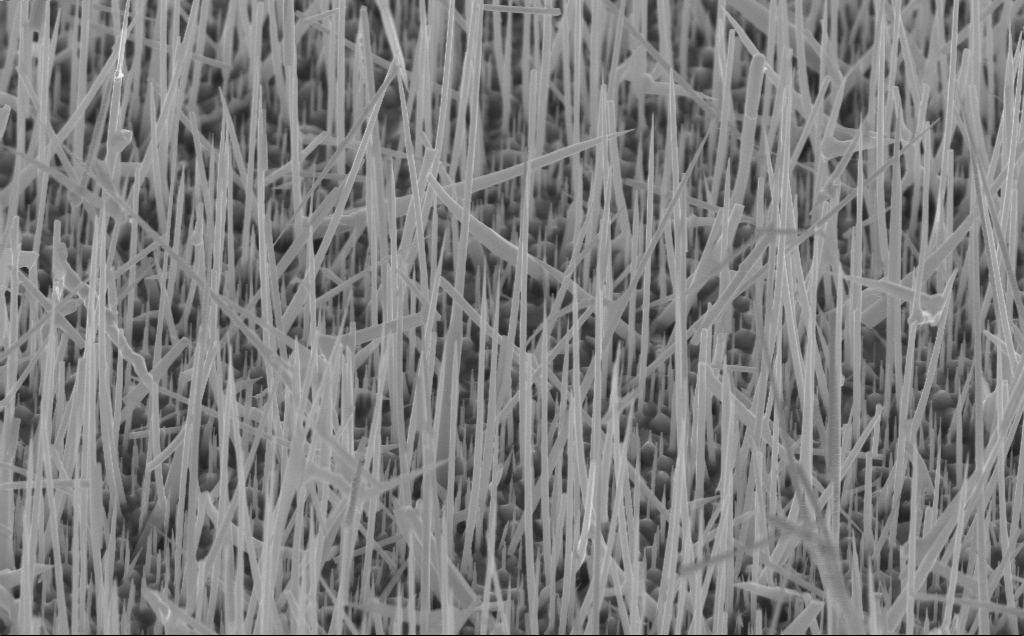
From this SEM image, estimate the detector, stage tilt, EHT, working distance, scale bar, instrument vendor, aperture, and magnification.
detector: InLens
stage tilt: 45°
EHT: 10 kV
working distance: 4 mm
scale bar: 1000 nm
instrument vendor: Zeiss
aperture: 30 µm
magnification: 20.78 K X